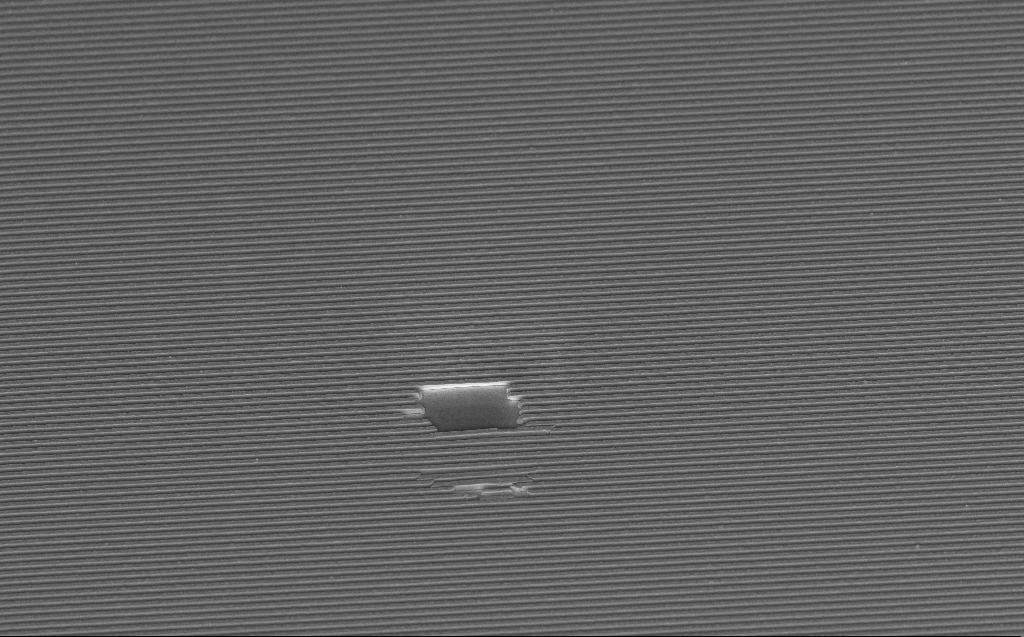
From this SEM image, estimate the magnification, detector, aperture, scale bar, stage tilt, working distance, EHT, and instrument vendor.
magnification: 5.72 K X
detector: InLens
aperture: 30 µm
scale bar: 10000 nm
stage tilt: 45°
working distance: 8 mm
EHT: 5 kV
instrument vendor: Zeiss